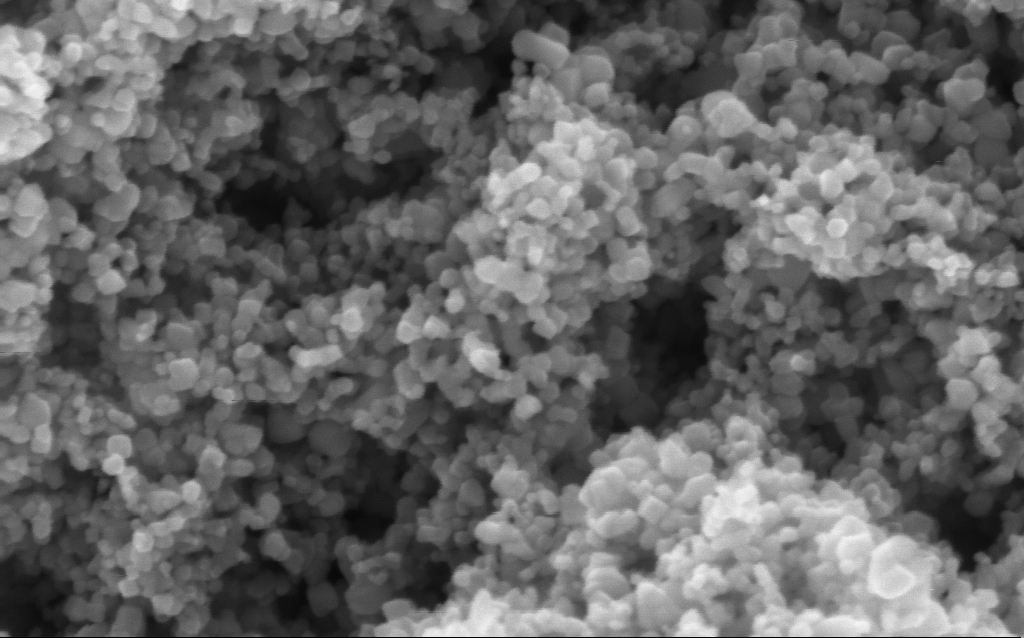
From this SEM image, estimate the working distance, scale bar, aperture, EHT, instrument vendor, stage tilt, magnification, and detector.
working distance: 4.5 mm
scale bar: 200 nm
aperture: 30 µm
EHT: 5 kV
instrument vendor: Zeiss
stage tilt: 0°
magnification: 294.6 K X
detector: InLens